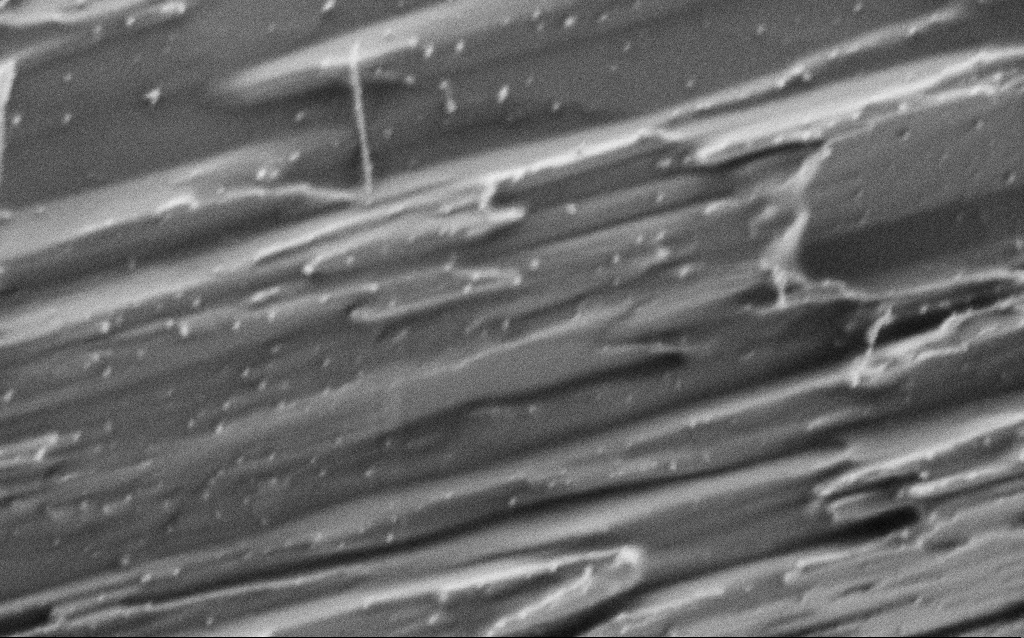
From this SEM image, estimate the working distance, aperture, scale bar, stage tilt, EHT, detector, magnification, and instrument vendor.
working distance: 4.3 mm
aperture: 30 µm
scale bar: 1000 nm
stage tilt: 0°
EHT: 0.8 kV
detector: SE2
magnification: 50 K X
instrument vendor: Zeiss